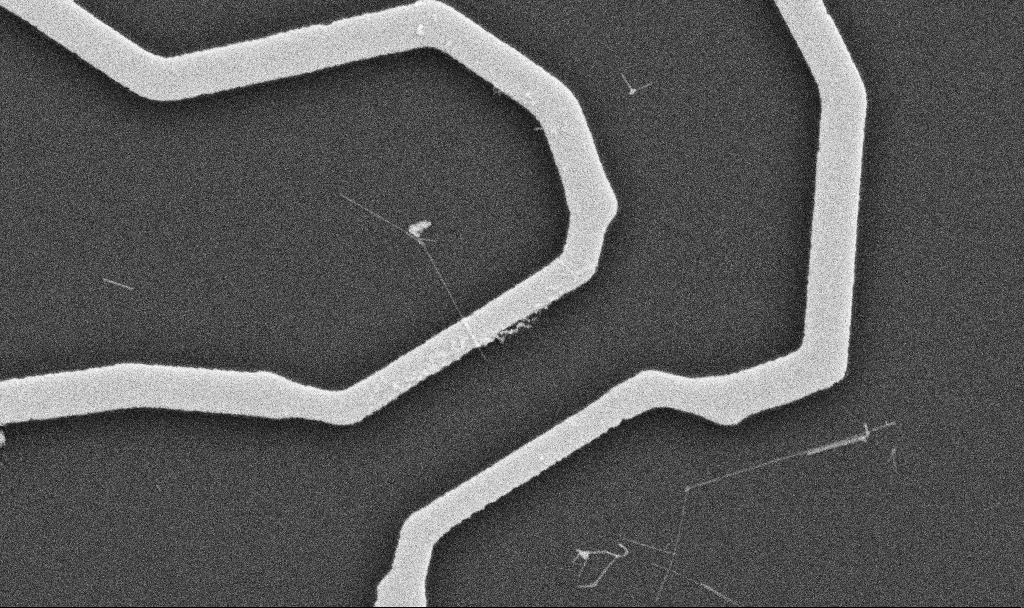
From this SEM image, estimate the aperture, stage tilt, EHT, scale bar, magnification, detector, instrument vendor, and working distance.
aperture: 30 µm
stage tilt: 0°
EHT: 10 kV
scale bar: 1000 nm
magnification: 20 K X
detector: SE2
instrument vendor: Zeiss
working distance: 10.7 mm